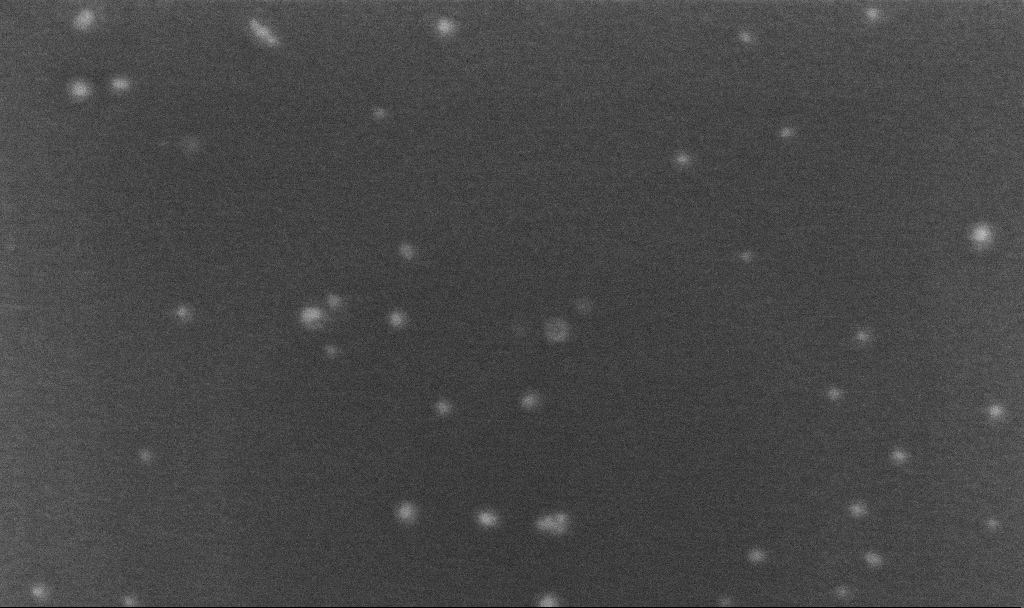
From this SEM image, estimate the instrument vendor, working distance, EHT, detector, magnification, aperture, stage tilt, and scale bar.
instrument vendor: Zeiss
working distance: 3.2 mm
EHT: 5 kV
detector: InLens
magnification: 400 K X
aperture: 30 µm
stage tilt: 0°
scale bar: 100 nm